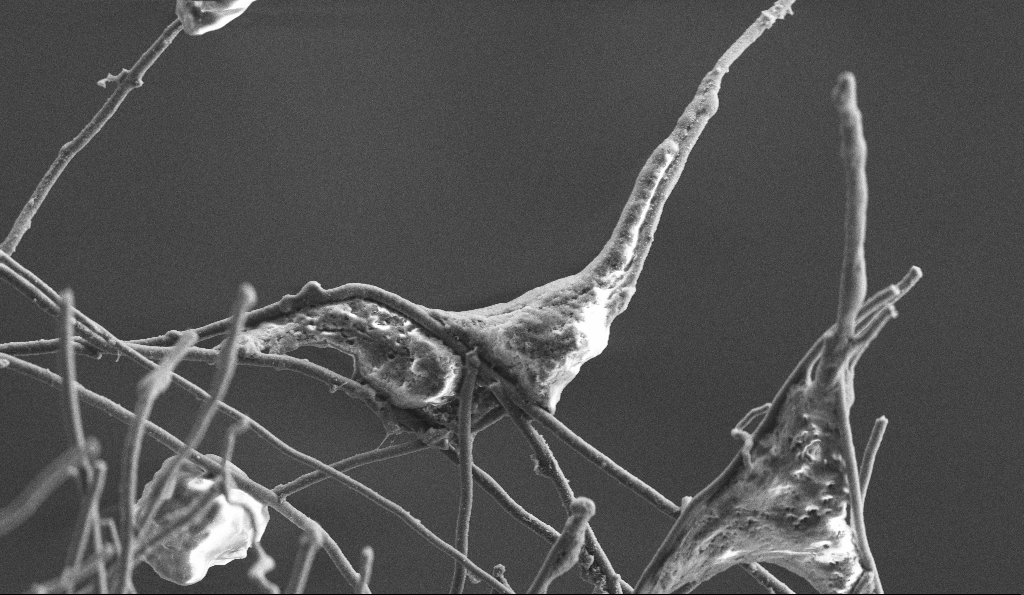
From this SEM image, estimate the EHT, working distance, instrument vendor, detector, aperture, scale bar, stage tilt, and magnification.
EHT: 3 kV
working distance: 5.9 mm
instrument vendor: Zeiss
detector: SE2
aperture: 30 µm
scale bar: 2000 nm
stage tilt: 0°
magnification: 8 K X